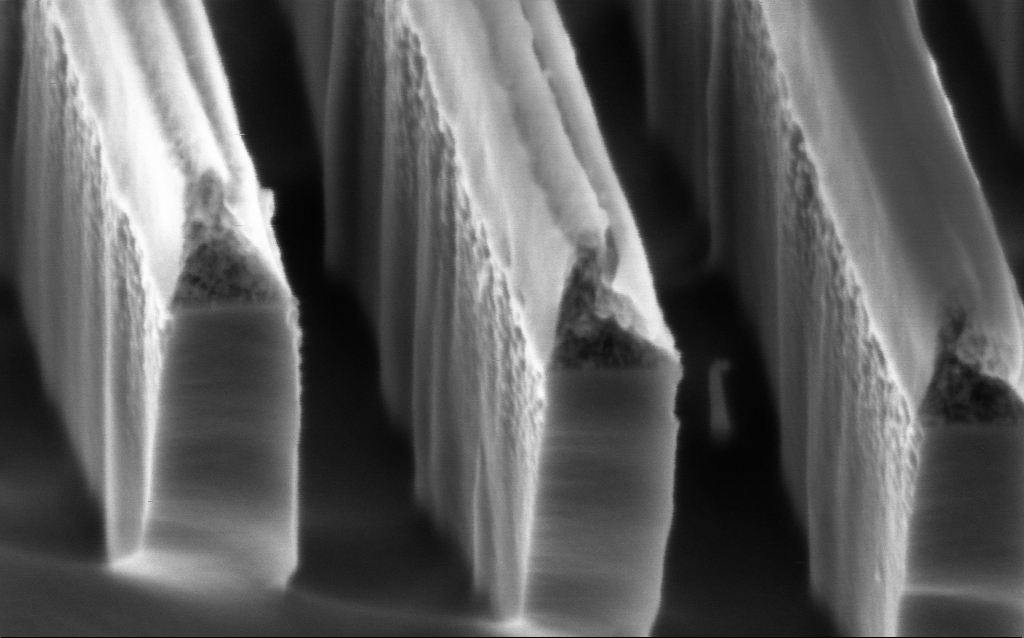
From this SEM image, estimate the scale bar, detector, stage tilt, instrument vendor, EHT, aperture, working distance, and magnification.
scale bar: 200 nm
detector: InLens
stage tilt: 45°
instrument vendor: Zeiss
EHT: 2 kV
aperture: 30 µm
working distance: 3.6 mm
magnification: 247.47 K X